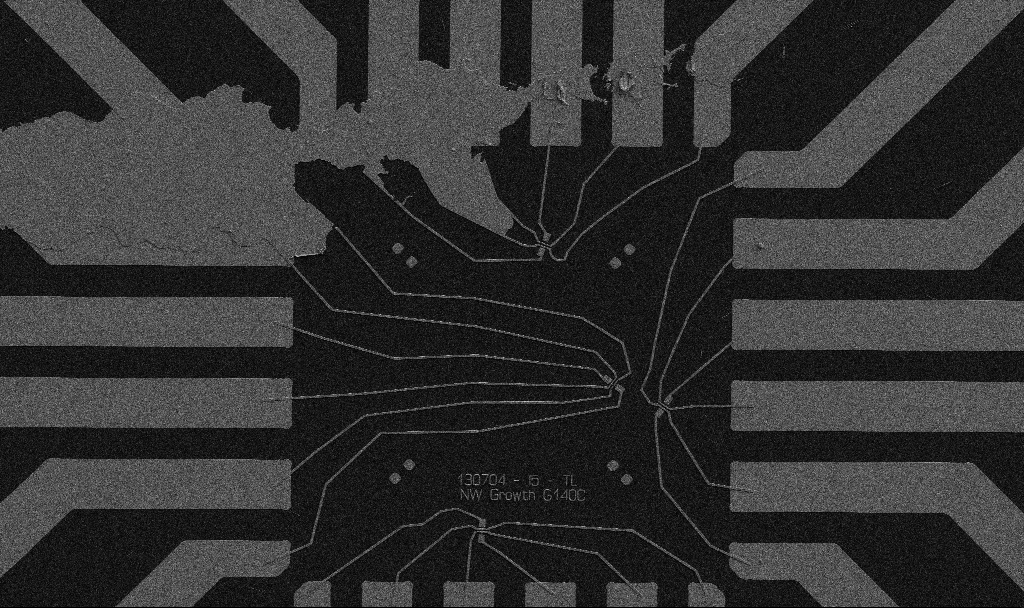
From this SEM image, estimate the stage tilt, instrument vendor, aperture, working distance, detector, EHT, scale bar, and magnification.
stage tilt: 0°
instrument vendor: Zeiss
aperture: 30 µm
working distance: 10.7 mm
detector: SE2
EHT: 5 kV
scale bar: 20000 nm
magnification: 1 K X